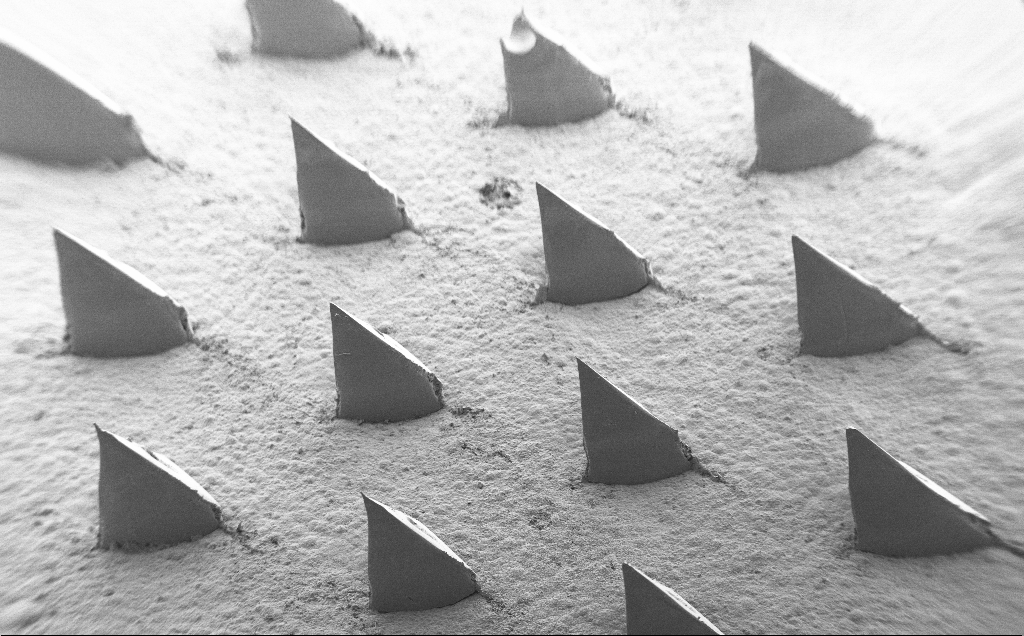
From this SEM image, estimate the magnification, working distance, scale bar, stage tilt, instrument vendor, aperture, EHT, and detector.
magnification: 0.069 K X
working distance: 9 mm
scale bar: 1e+06 nm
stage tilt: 40°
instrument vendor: Zeiss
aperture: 30 µm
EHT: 5 kV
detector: SE2